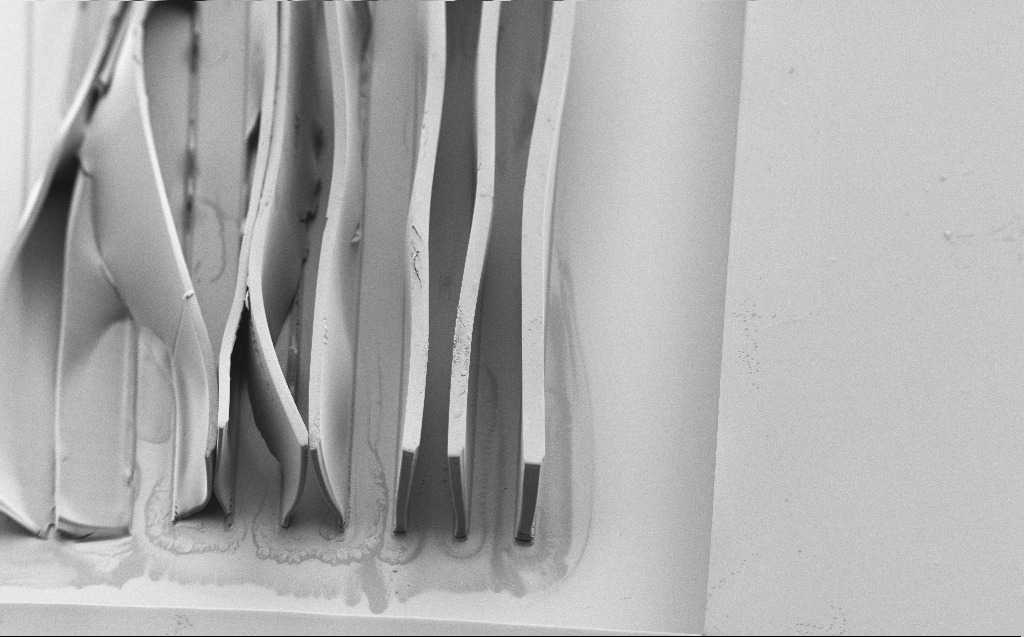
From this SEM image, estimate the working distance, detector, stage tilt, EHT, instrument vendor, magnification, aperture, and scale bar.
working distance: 6 mm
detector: SE2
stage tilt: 45°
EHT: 1.2 kV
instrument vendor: Zeiss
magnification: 0.344 K X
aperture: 30 µm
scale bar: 100000 nm